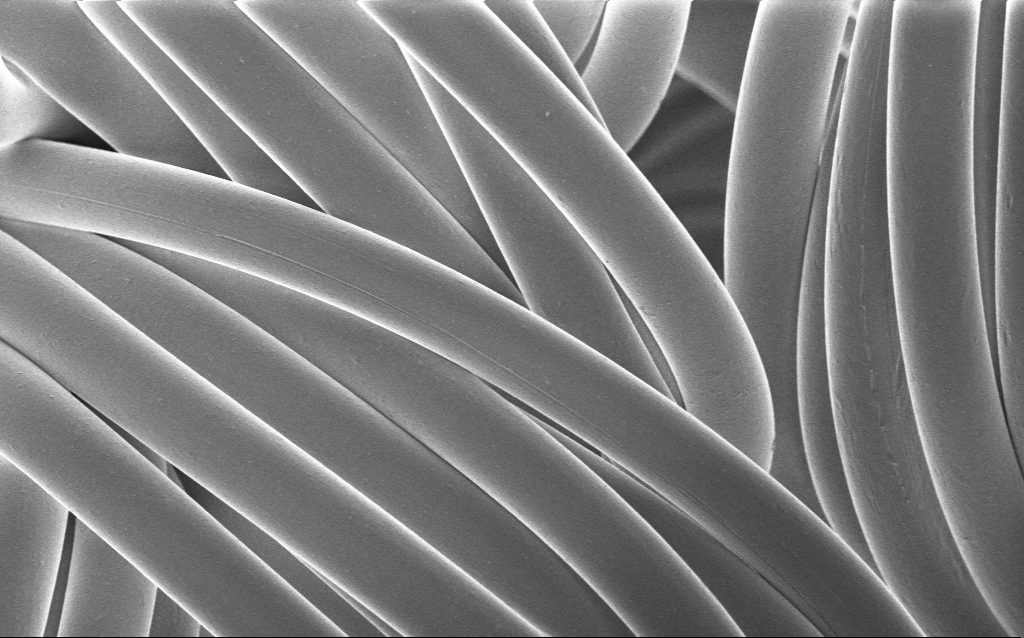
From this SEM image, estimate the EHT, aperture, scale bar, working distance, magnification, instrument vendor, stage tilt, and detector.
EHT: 1 kV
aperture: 30 µm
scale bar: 10000 nm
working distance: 4 mm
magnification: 1.35 K X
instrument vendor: Zeiss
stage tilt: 0°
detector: InLens